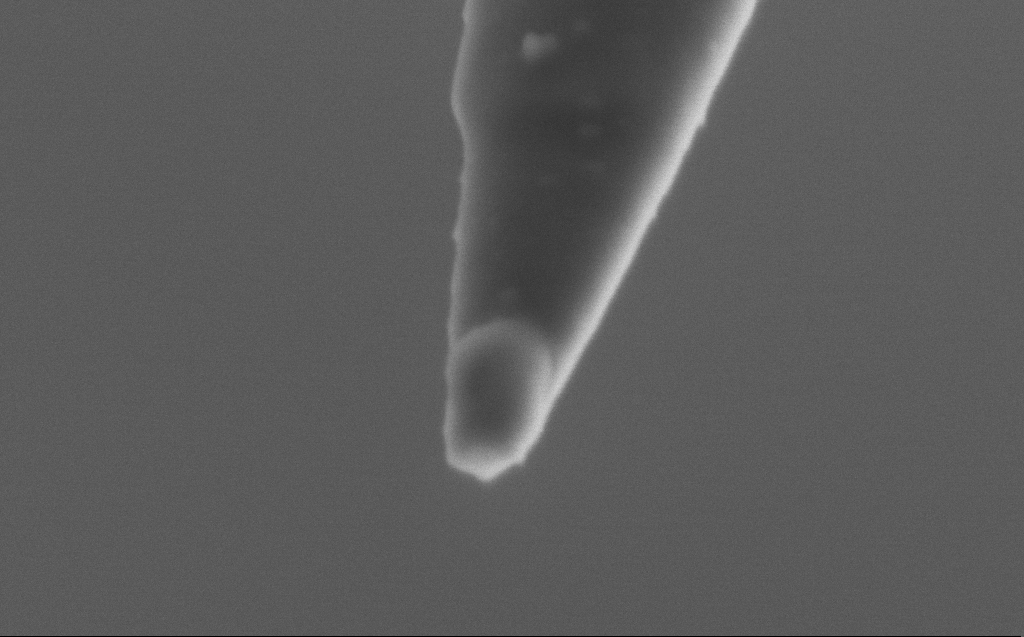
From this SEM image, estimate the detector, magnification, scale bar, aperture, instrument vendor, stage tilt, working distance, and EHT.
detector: SE2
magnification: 250 K X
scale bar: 200 nm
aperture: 30 µm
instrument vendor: Zeiss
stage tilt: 45°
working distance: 5 mm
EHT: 2 kV